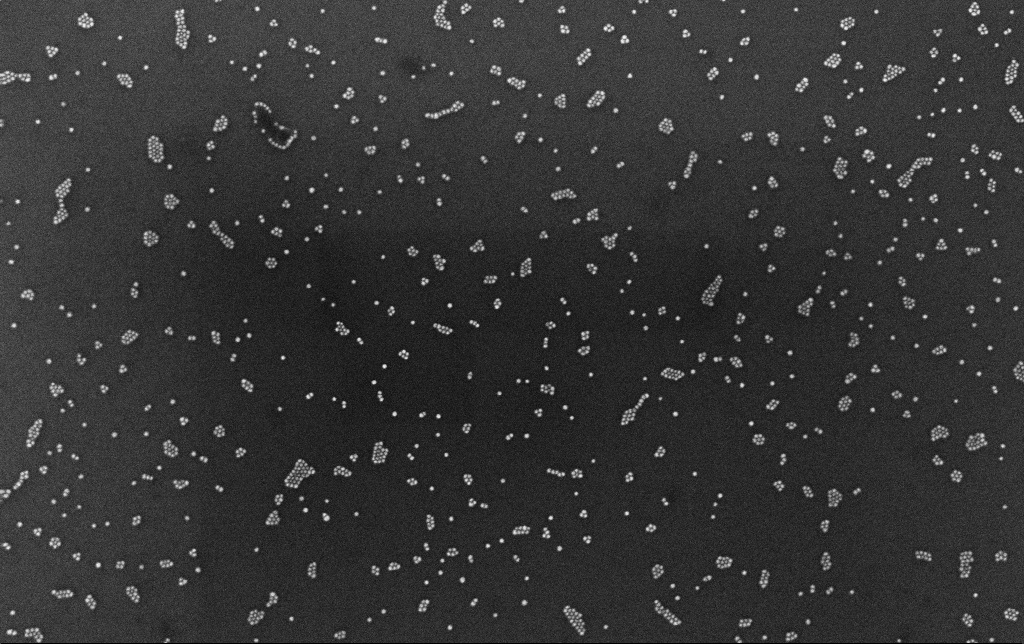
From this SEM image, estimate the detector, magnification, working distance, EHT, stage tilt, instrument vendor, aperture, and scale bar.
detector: InLens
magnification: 100 K X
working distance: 3.4 mm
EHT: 10 kV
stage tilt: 0°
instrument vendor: Zeiss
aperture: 30 µm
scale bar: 200 nm